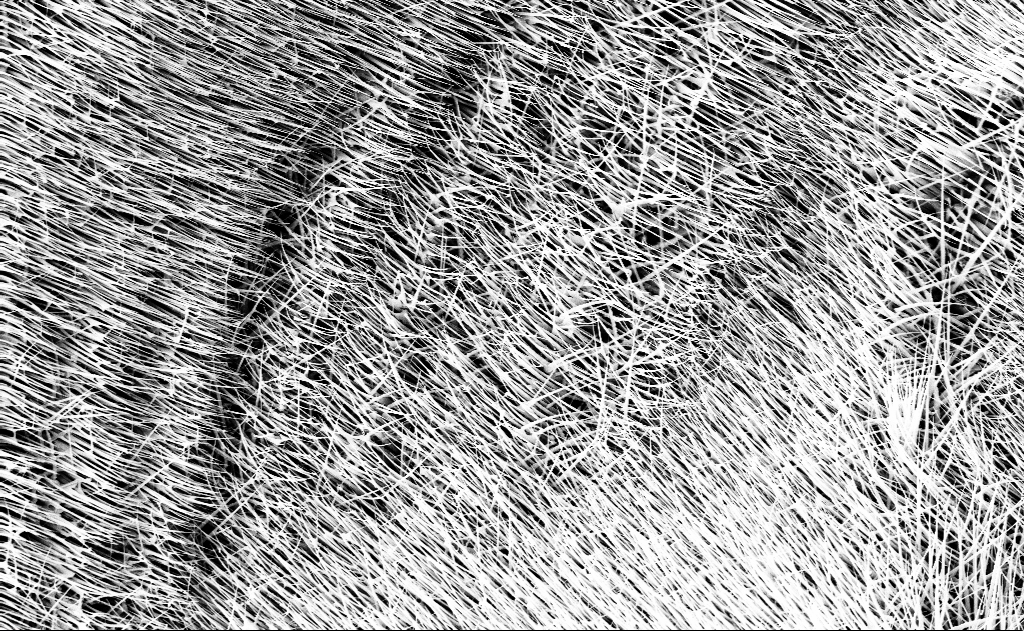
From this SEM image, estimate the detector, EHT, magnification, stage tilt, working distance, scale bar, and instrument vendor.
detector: InLens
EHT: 10 kV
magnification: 10 K X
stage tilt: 0°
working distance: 13 mm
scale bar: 2000 nm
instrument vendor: Zeiss